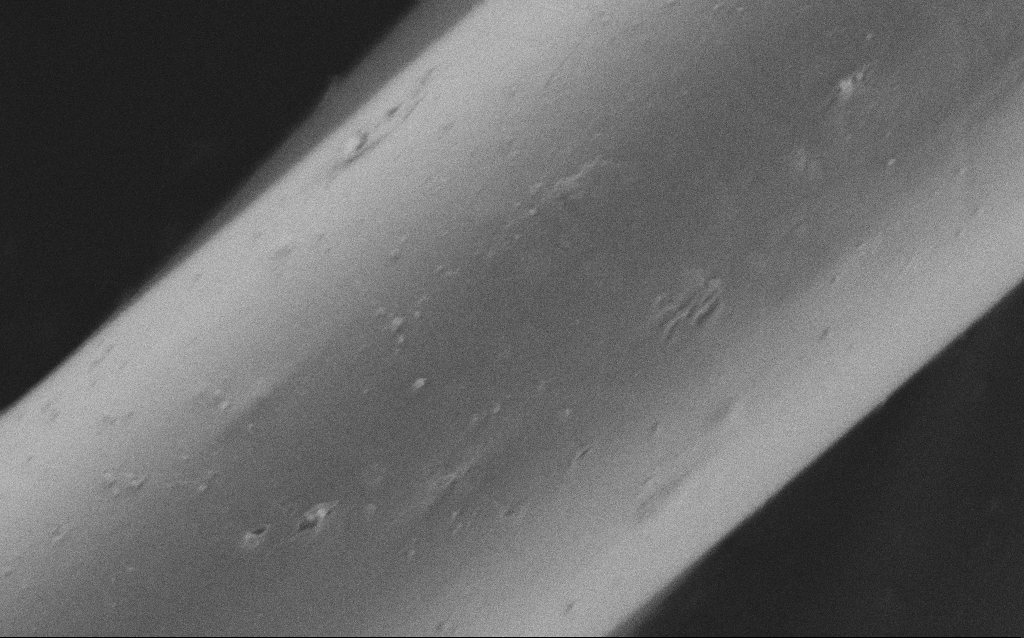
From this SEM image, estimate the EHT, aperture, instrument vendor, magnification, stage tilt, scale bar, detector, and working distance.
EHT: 1 kV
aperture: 30 µm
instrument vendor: Zeiss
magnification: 16.4 K X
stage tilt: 0°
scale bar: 1000 nm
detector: SE2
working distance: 5 mm